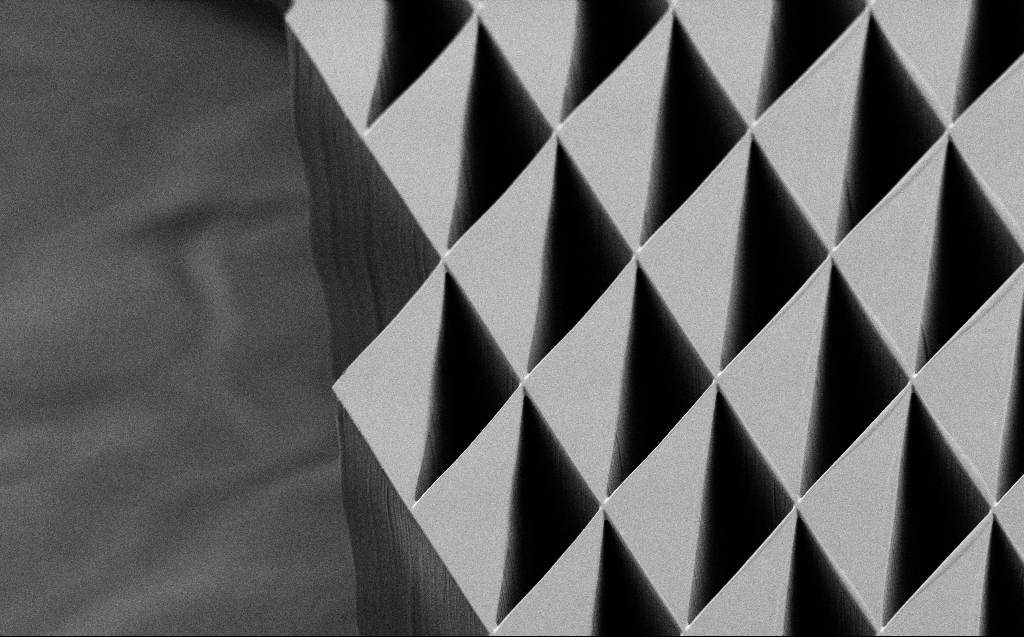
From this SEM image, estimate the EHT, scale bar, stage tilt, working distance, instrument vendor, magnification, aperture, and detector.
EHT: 1 kV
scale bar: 20000 nm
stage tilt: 45°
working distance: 5 mm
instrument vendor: Zeiss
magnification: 0.995 K X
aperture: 30 µm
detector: SE2